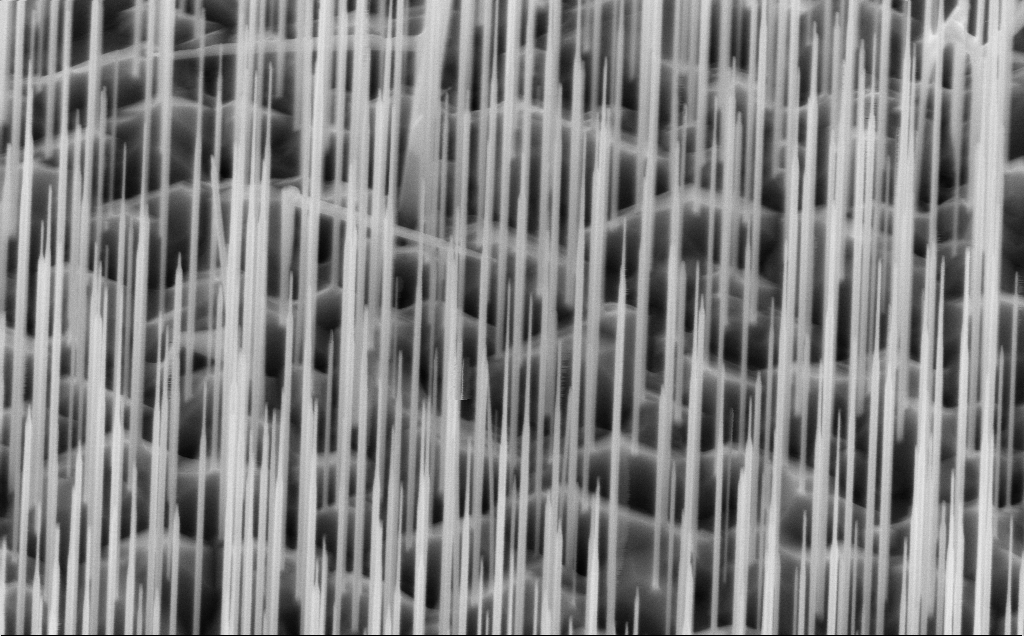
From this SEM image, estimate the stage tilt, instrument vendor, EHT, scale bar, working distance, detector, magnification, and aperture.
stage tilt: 45°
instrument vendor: Zeiss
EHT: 10 kV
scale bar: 1000 nm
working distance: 5 mm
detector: InLens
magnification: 40 K X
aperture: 30 µm